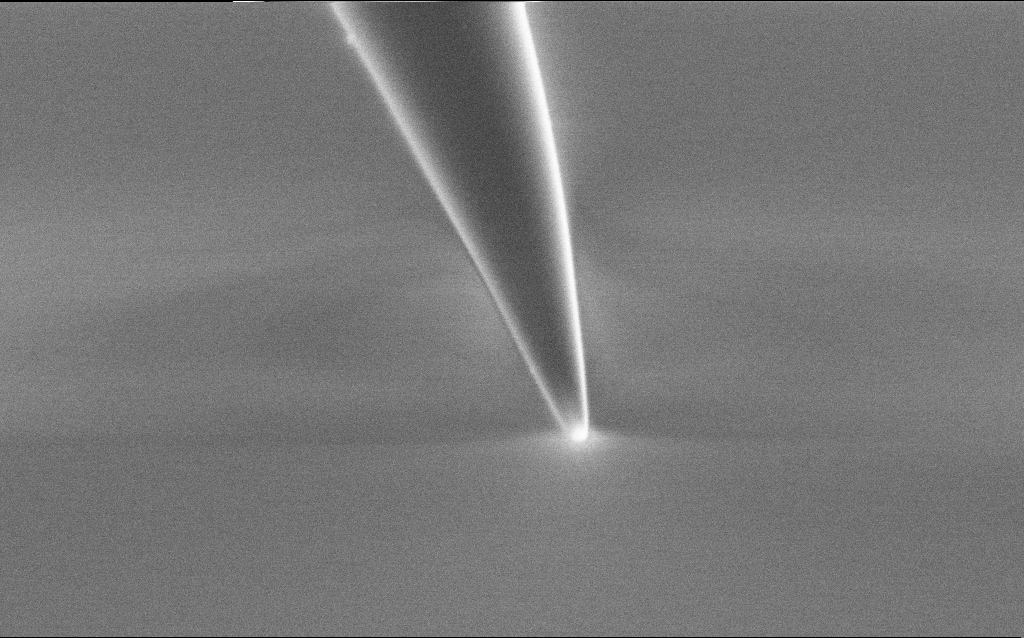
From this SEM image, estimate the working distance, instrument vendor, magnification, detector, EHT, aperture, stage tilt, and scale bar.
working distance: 7 mm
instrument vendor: Zeiss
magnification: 50 K X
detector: SE2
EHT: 1 kV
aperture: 30 µm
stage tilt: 45°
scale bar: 1000 nm